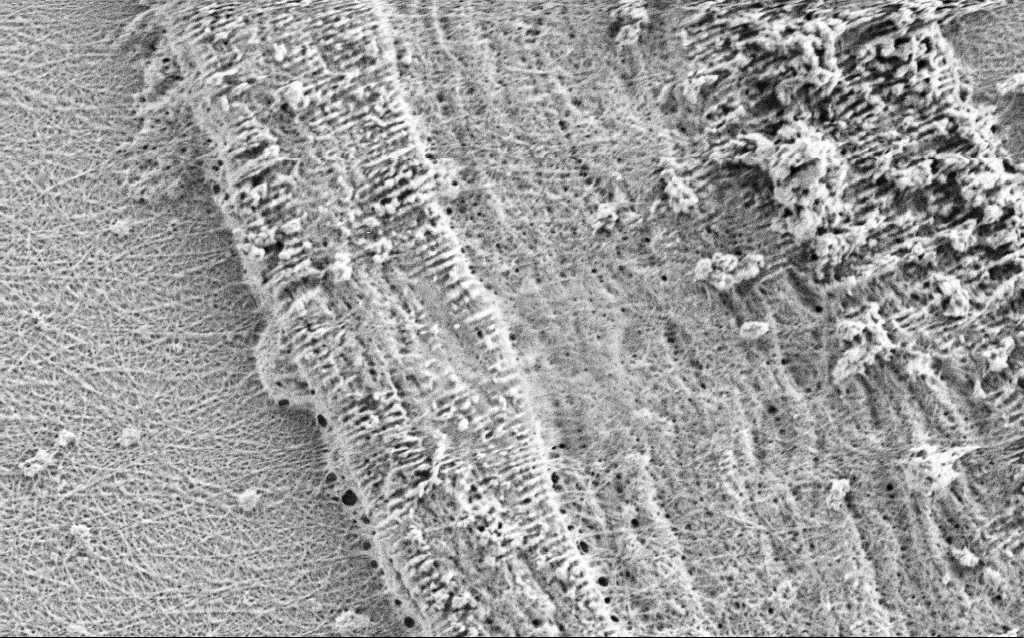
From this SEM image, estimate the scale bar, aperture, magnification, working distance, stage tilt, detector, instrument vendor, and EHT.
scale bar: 2000 nm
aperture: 30 µm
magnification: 20 K X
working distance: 3 mm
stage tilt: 0°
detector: SE2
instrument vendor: Zeiss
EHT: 0.9 kV